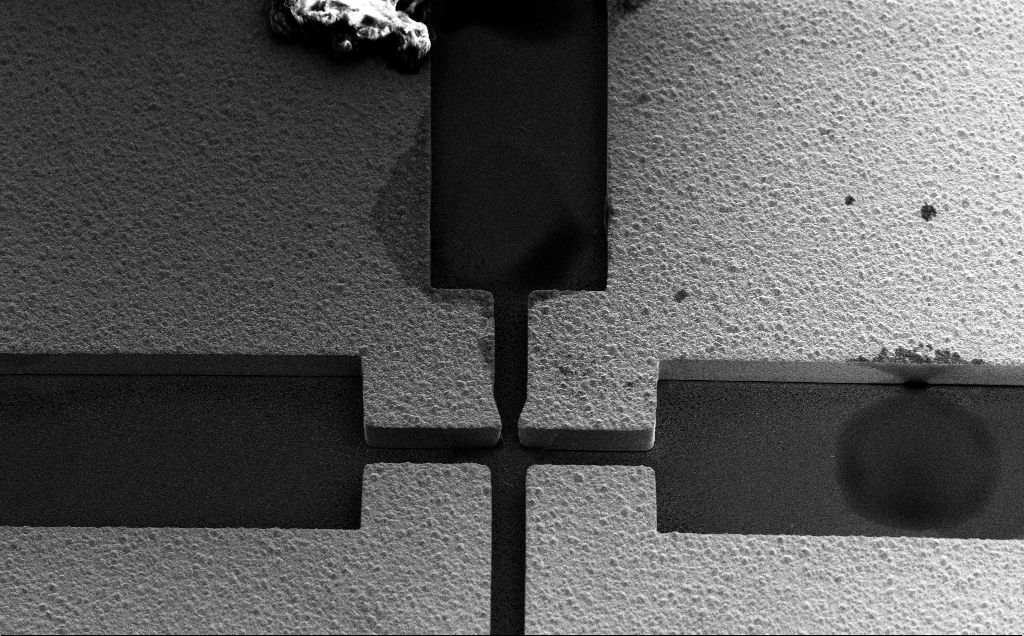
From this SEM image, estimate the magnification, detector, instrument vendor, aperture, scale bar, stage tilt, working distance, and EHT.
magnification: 1.33 K X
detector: SE2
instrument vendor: Zeiss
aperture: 30 µm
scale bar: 20000 nm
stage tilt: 45°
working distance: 17 mm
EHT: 10 kV